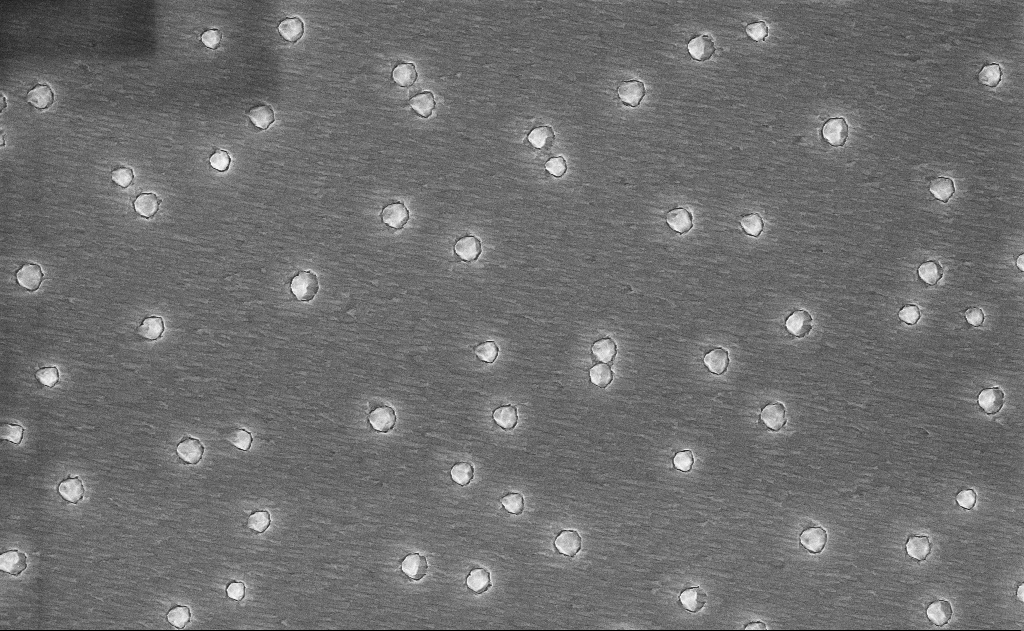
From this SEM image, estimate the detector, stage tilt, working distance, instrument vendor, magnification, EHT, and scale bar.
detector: InLens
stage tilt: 0°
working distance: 16 mm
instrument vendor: Zeiss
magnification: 20 K X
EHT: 10 kV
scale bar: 2000 nm